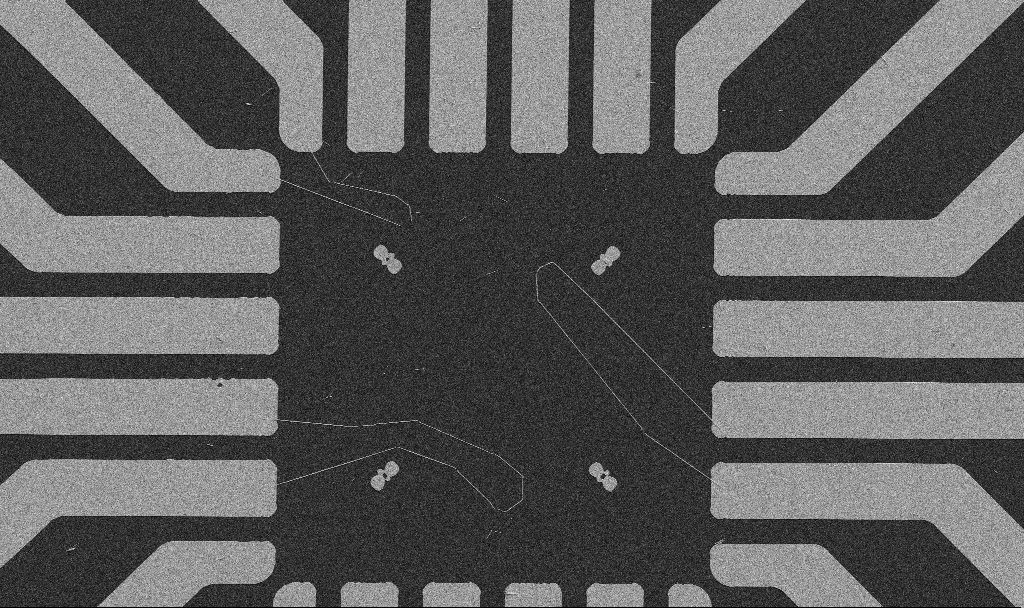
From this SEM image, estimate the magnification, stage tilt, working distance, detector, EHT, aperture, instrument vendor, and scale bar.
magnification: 1 K X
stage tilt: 0°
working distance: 10.7 mm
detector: SE2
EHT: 5 kV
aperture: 30 µm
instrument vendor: Zeiss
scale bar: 20000 nm